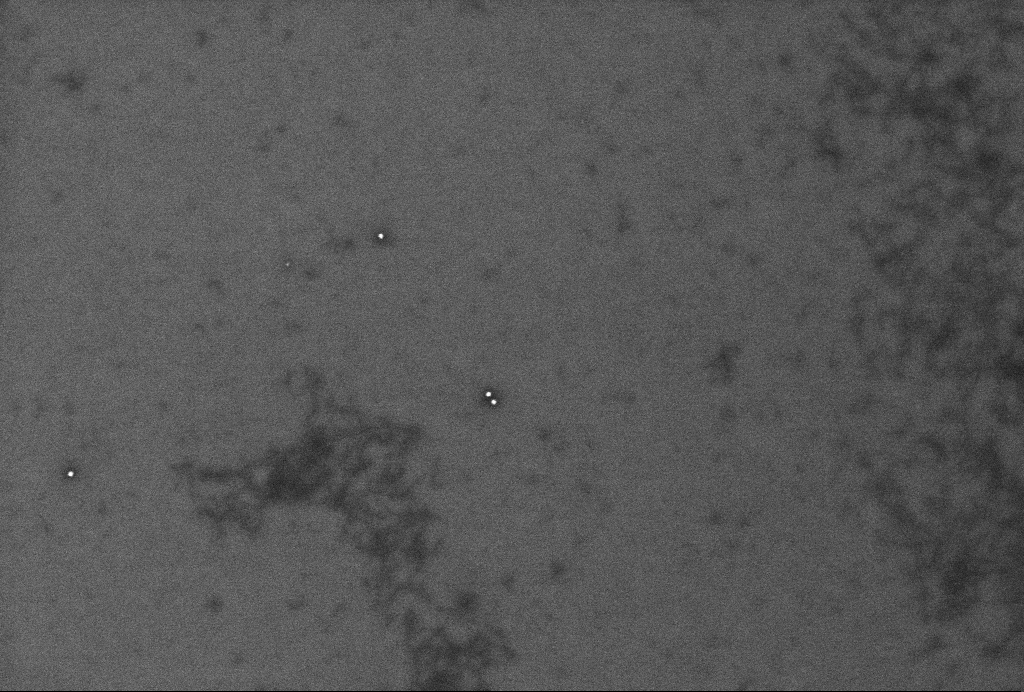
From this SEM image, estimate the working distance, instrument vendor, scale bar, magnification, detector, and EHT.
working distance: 3.3 mm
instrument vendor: Zeiss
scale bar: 200 nm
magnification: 62.65 K X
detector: InLens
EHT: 2 kV